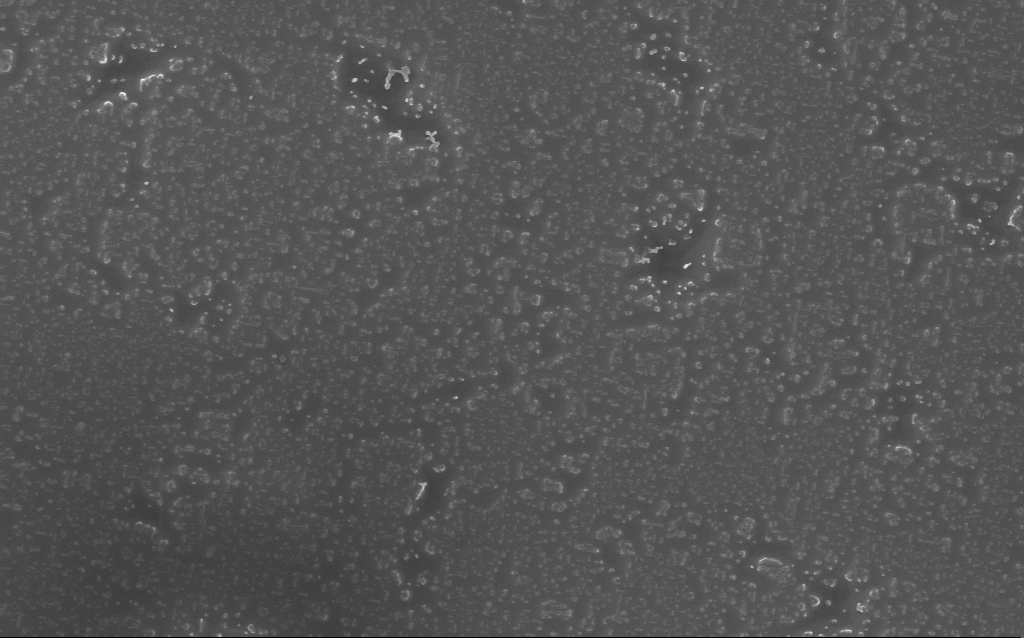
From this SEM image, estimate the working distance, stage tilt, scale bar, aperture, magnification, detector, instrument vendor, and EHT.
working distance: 5 mm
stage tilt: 0°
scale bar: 1000 nm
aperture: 30 µm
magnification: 65.21 K X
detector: InLens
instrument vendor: Zeiss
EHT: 10 kV